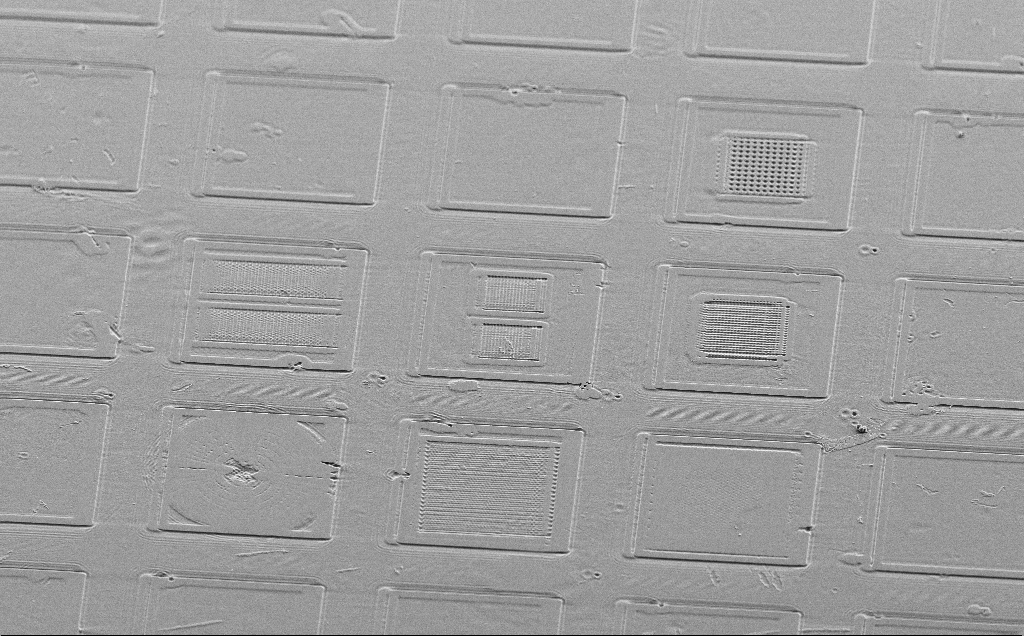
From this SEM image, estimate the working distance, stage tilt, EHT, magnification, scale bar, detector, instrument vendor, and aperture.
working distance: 10 mm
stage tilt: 45°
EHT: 5 kV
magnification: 0.619 K X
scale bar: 100000 nm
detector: SE2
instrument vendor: Zeiss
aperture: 30 µm